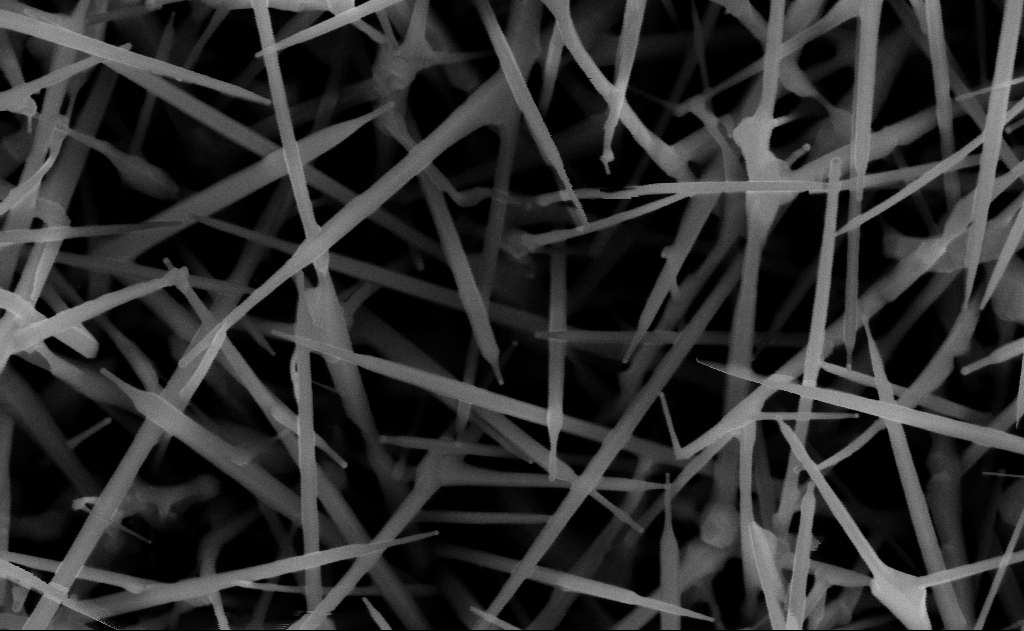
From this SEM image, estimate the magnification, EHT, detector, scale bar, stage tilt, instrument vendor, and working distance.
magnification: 80 K X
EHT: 10 kV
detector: InLens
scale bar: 200 nm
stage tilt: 0°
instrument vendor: Zeiss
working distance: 13 mm